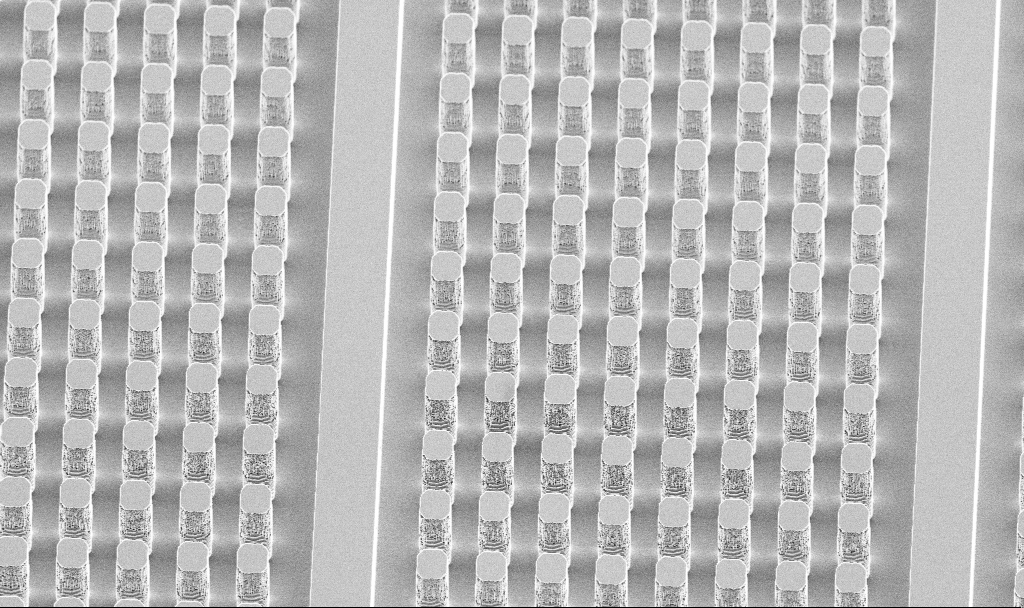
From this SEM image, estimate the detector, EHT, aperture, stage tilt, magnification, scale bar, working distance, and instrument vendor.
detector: SE2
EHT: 5 kV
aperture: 30 µm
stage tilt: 45°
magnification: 1.09 K X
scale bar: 20000 nm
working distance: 16.6 mm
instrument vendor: Zeiss